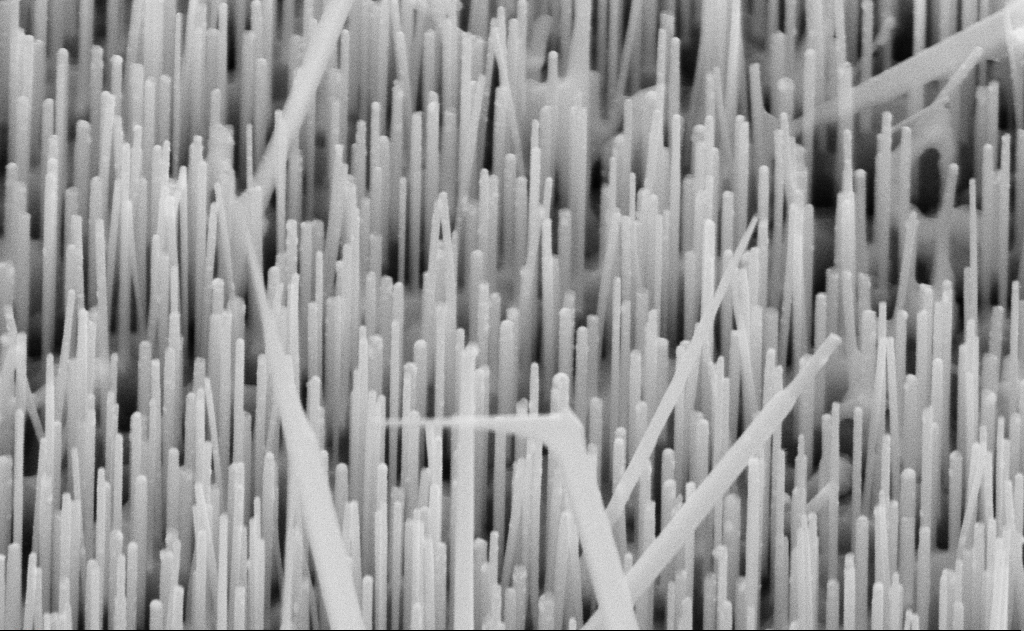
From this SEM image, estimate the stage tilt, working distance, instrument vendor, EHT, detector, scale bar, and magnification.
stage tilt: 45°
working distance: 11 mm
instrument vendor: Zeiss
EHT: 10 kV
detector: SE2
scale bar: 200 nm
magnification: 80 K X